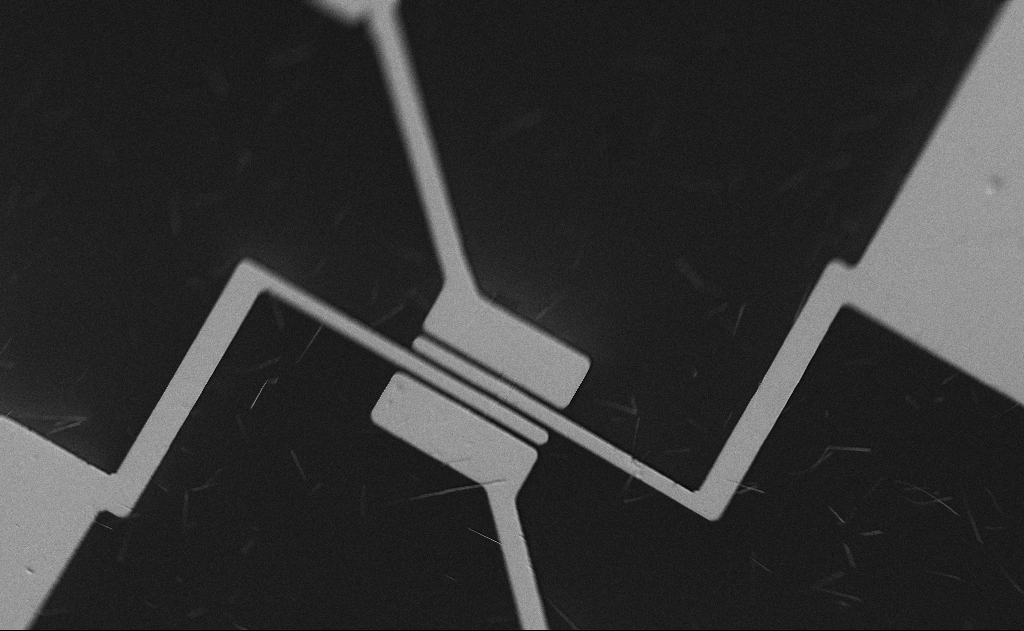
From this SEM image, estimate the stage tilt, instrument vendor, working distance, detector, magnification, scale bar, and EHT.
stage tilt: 0°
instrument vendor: Zeiss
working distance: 21 mm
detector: SE2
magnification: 2 K X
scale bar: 10000 nm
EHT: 5 kV